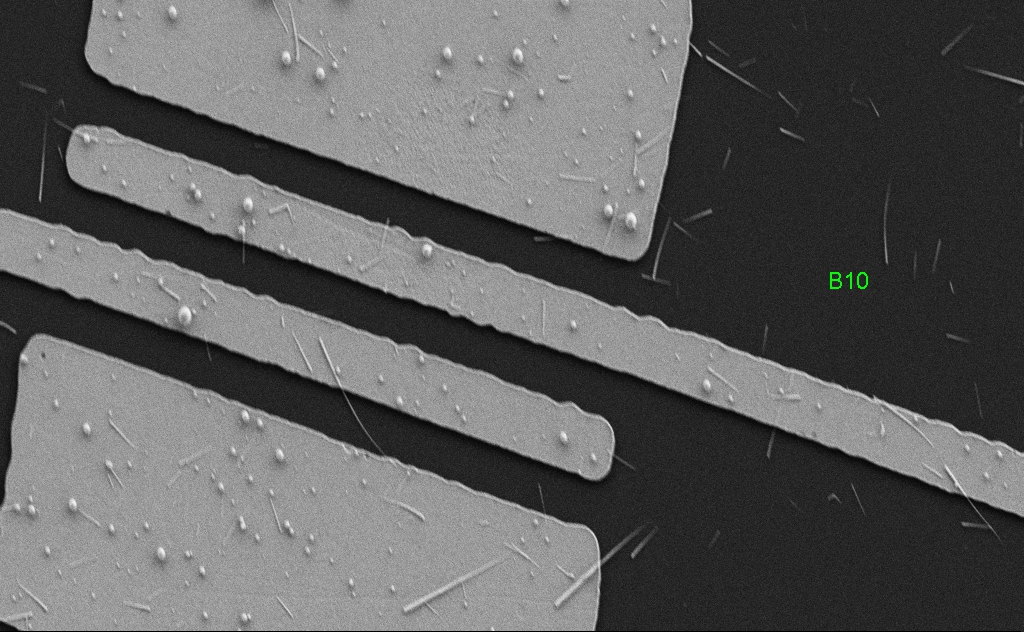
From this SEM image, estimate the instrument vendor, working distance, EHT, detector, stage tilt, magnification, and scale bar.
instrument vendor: Zeiss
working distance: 5 mm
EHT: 5 kV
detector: SE2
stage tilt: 0°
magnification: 7.54 K X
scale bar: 2000 nm